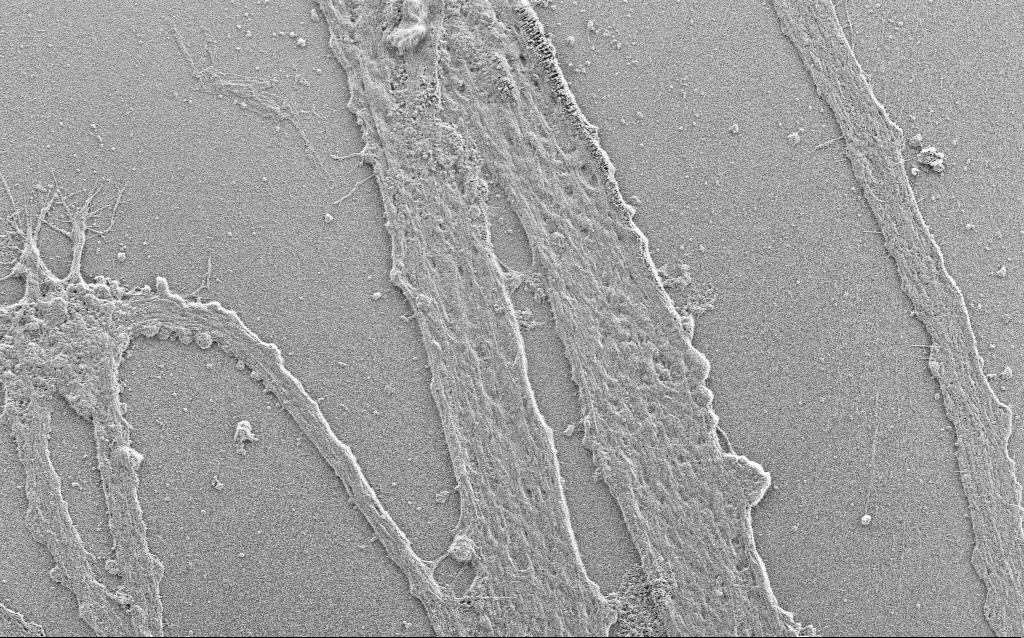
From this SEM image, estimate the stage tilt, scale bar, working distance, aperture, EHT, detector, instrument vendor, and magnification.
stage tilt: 0°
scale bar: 20000 nm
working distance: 4 mm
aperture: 30 µm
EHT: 0.9 kV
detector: SE2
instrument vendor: Zeiss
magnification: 2.5 K X